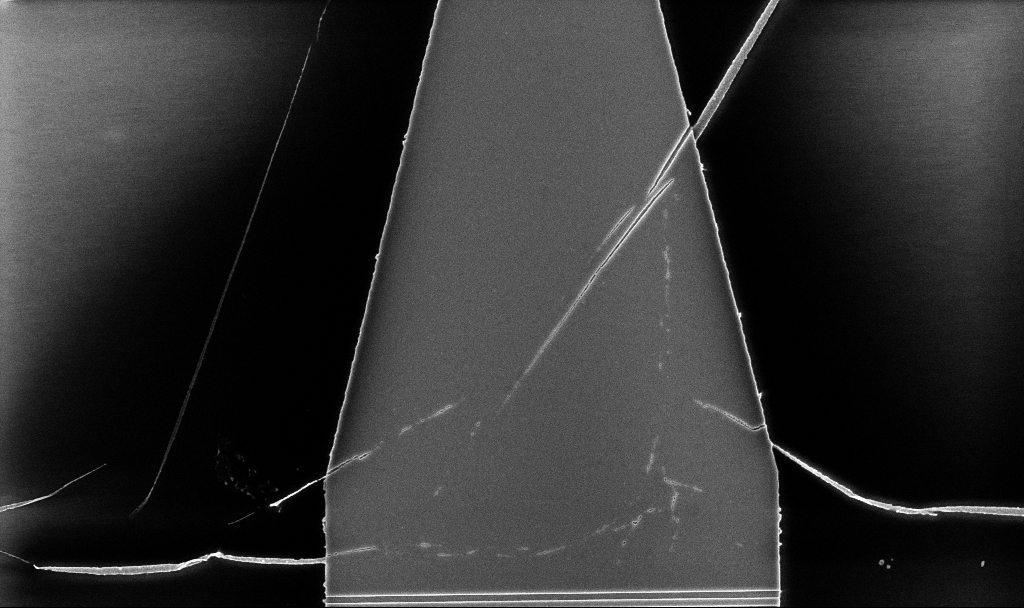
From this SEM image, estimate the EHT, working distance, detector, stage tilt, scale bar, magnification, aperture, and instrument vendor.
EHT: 5 kV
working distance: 5.2 mm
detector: InLens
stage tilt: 0°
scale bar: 2000 nm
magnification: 8.45 K X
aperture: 30 µm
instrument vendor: Zeiss